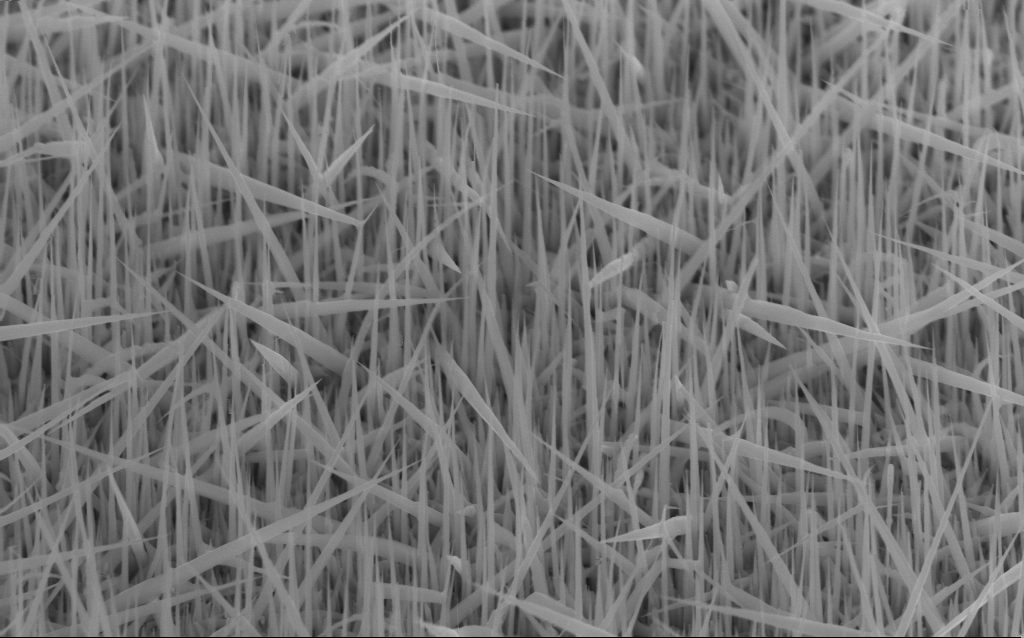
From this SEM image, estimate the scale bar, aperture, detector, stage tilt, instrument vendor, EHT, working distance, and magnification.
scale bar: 1000 nm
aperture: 30 µm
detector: InLens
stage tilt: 45.1°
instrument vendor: Zeiss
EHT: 10 kV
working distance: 5 mm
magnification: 42.11 K X